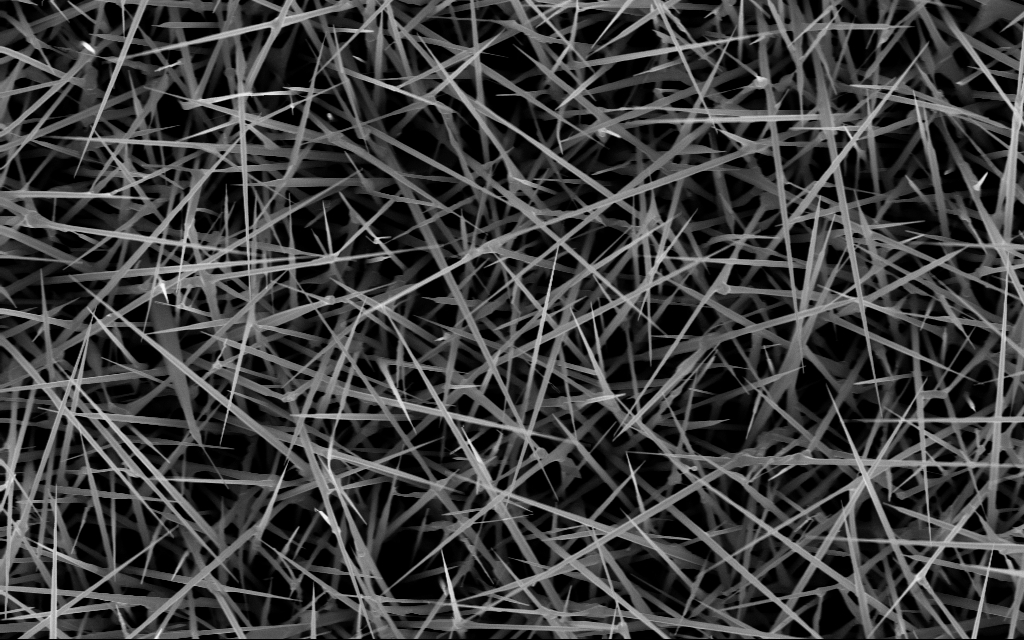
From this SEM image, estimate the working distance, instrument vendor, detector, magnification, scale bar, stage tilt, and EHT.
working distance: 7 mm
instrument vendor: Zeiss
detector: InLens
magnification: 20 K X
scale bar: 2000 nm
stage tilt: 0°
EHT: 10 kV